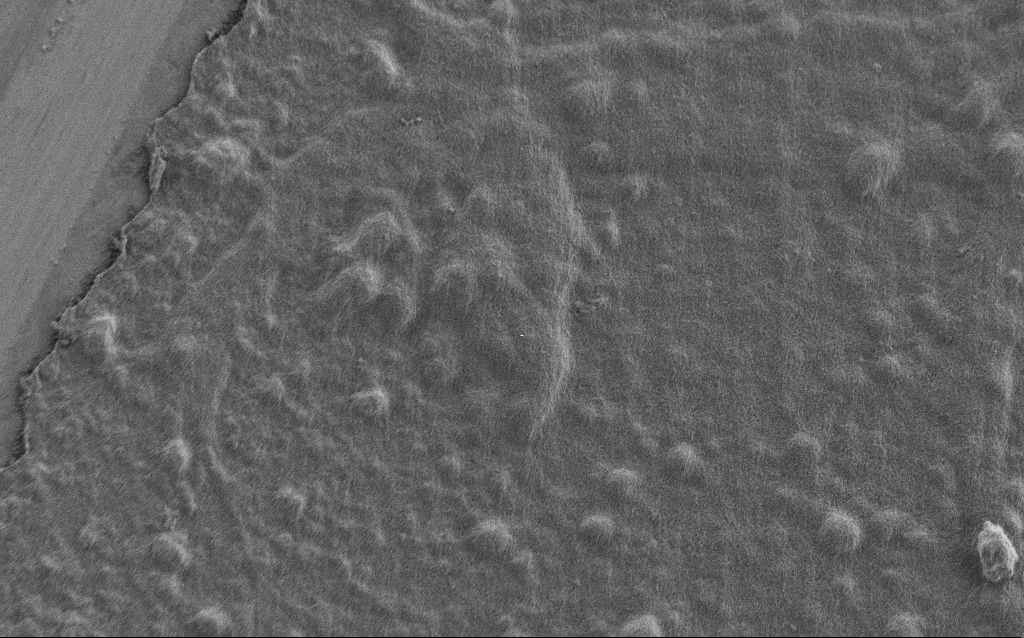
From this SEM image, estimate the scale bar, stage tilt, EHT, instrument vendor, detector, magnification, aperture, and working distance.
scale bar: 10000 nm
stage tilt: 0°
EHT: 0.9 kV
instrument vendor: Zeiss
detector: SE2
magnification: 5 K X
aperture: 30 µm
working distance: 7 mm